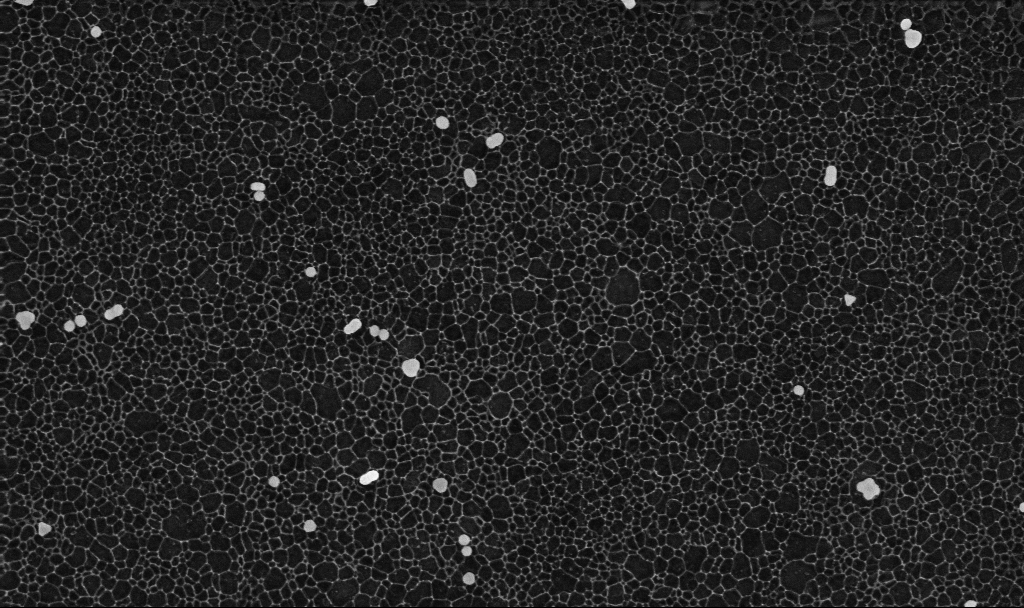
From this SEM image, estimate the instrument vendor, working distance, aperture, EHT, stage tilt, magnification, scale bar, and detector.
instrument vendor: Zeiss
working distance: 3.4 mm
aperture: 30 µm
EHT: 10 kV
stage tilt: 0°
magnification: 70 K X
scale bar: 1000 nm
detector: InLens